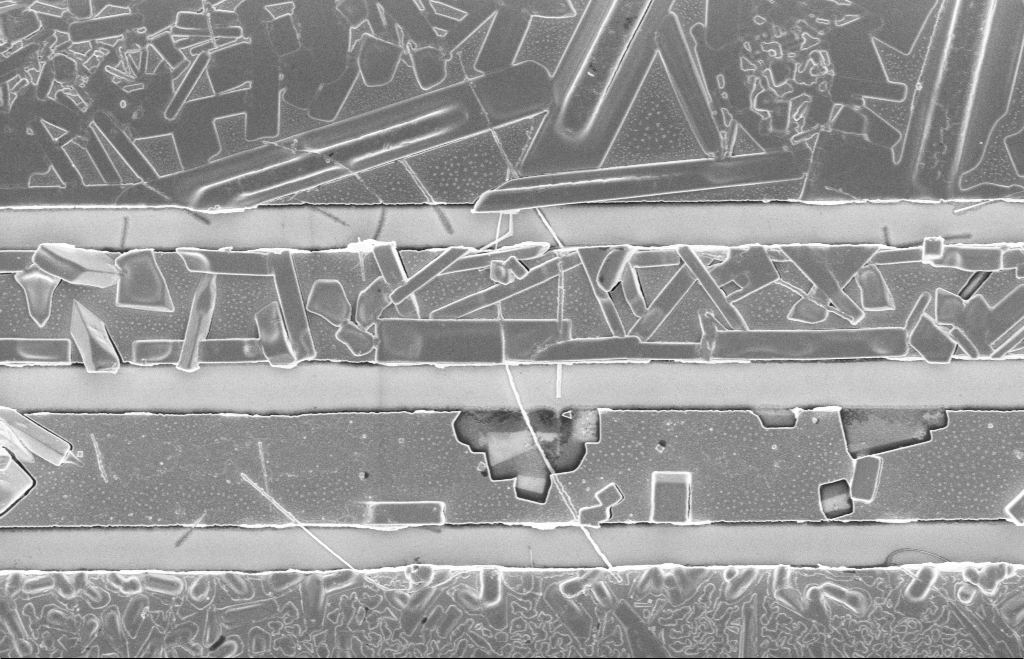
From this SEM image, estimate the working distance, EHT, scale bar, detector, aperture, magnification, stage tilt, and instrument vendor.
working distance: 8 mm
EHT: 5 kV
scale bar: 1000 nm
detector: InLens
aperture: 20 µm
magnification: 9.94 K X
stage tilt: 0°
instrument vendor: Zeiss